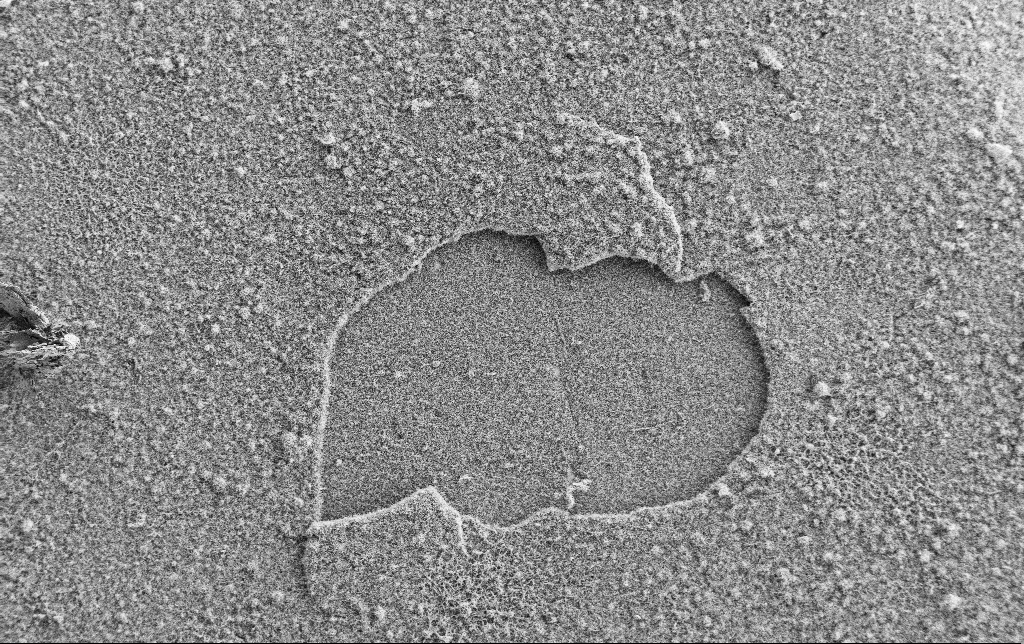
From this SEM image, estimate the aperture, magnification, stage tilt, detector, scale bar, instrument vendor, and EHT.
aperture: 30 µm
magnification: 0.5 K X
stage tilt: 0°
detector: SE2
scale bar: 100000 nm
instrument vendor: Zeiss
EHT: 2 kV